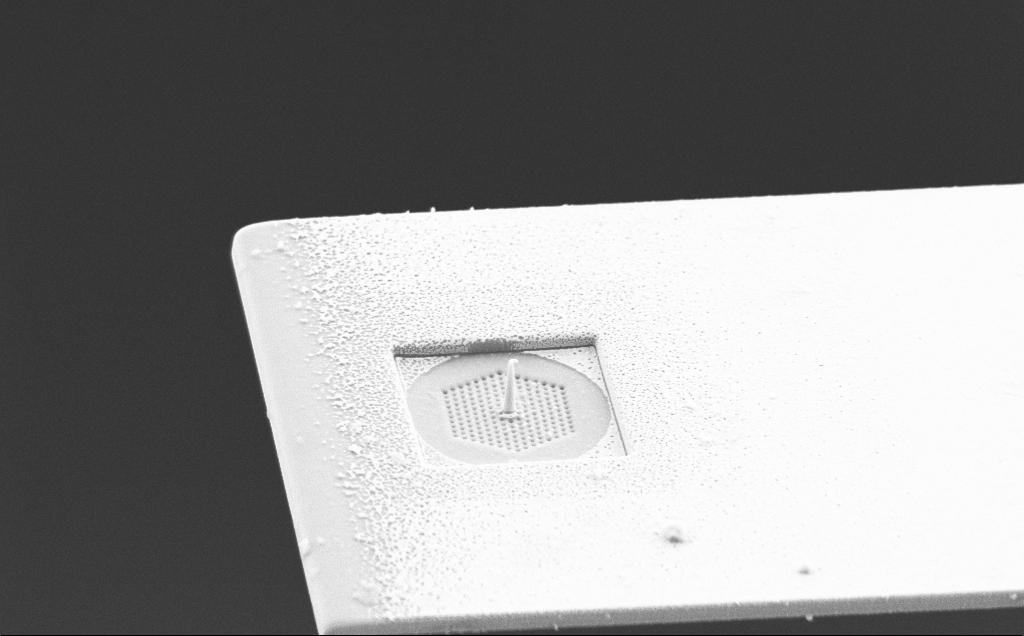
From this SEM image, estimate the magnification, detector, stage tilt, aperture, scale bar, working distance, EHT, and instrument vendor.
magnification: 12.47 K X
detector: SE2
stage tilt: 53.9°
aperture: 30 µm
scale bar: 2000 nm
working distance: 6 mm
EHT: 5 kV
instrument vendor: Zeiss